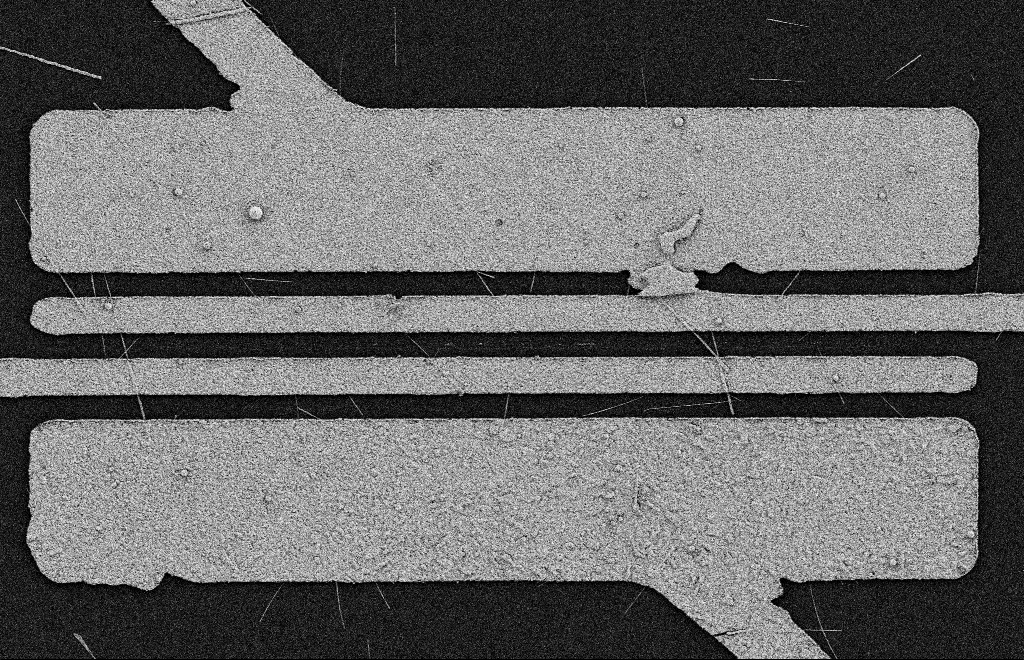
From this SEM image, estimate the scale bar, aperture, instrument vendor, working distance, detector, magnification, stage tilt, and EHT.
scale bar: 2000 nm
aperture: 20 µm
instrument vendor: Zeiss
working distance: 11 mm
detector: SE2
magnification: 5.66 K X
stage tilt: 0°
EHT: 2 kV